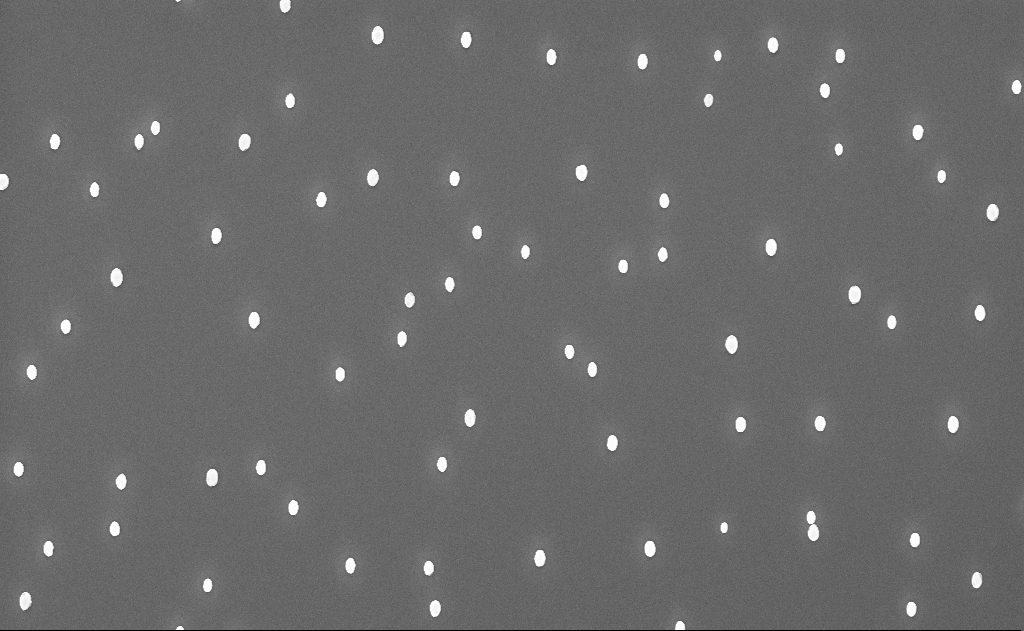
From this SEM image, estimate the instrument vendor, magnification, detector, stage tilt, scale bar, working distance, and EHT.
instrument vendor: Zeiss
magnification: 10 K X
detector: InLens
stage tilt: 0°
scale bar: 2000 nm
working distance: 12 mm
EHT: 10 kV